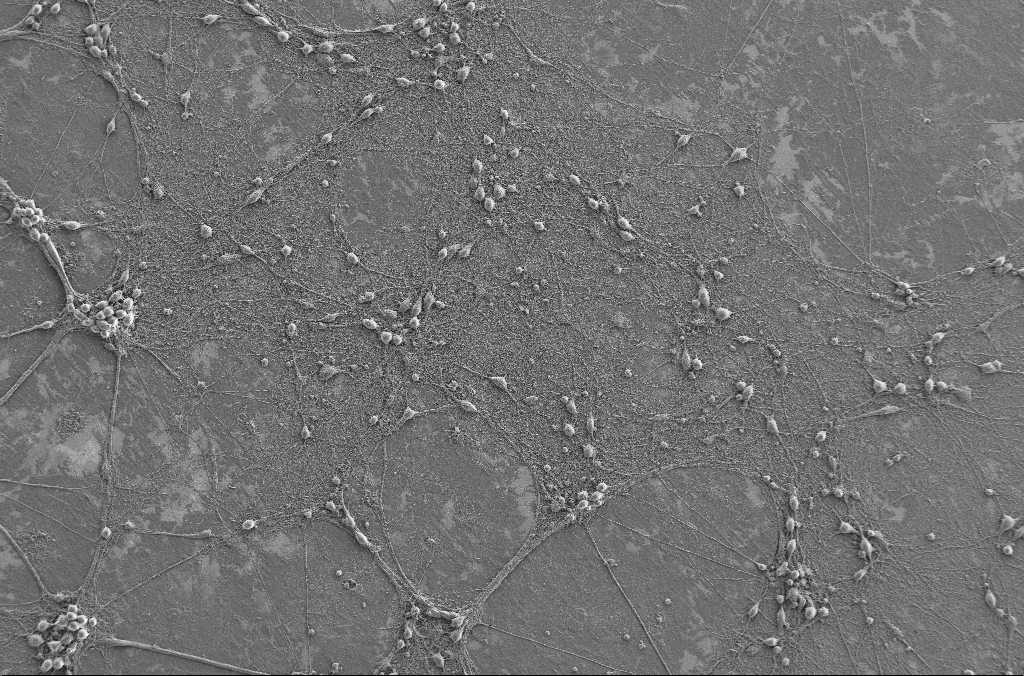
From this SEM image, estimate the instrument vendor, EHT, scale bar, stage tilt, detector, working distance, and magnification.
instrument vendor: Zeiss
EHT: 2 kV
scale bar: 100000 nm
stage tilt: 0°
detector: SE2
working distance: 4.1 mm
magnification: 0.5 K X